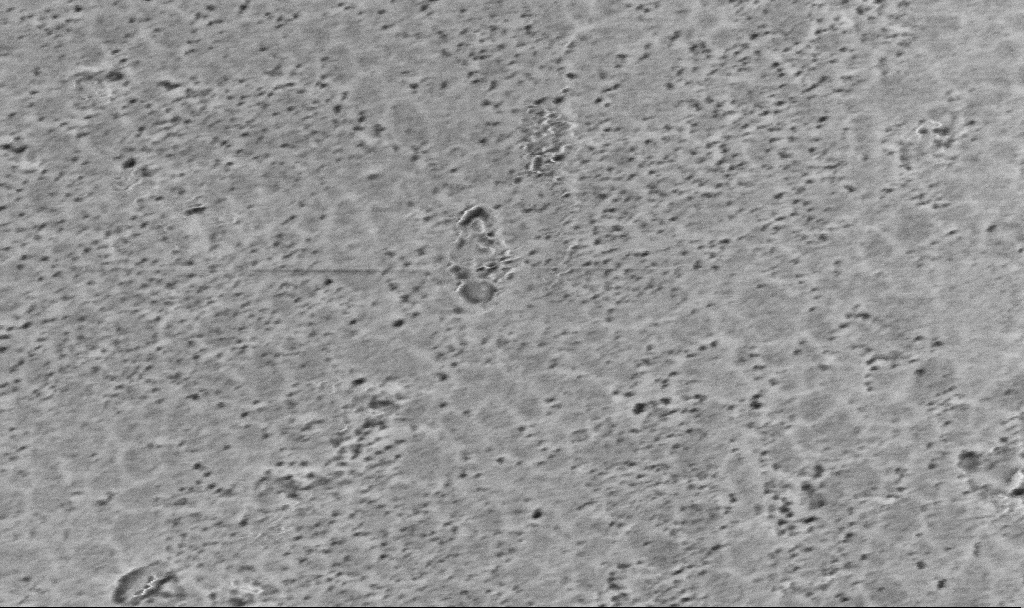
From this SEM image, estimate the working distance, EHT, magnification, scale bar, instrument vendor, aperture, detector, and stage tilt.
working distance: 7 mm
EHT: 2 kV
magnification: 100.42 K X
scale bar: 200 nm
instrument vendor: Zeiss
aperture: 30 µm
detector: InLens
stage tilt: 0°